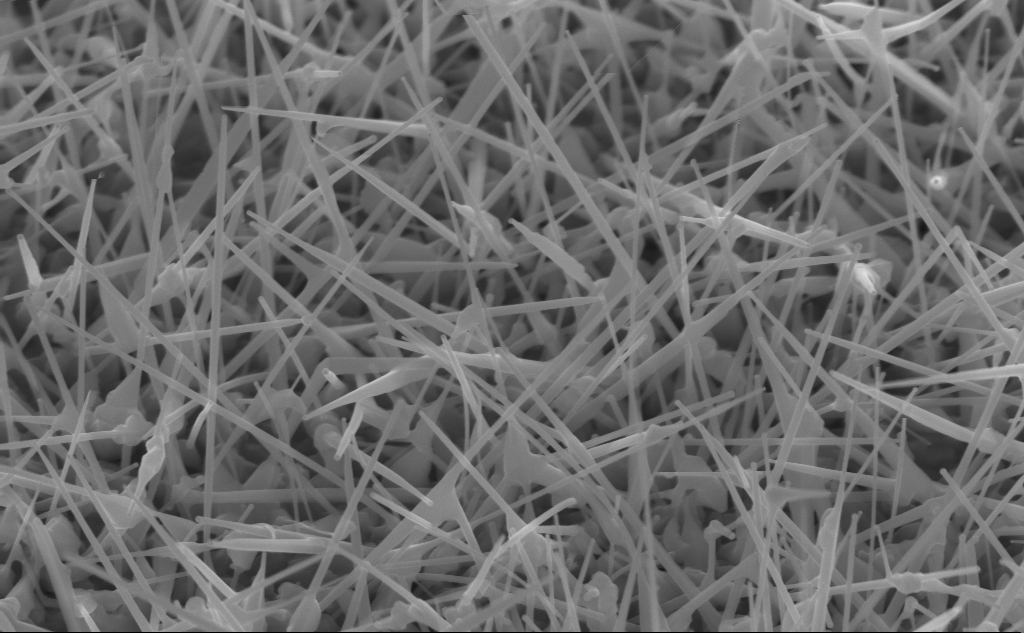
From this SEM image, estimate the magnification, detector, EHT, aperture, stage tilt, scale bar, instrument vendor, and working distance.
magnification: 53.52 K X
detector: InLens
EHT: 10 kV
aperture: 30 µm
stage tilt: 45°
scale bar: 1000 nm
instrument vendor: Zeiss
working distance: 4 mm